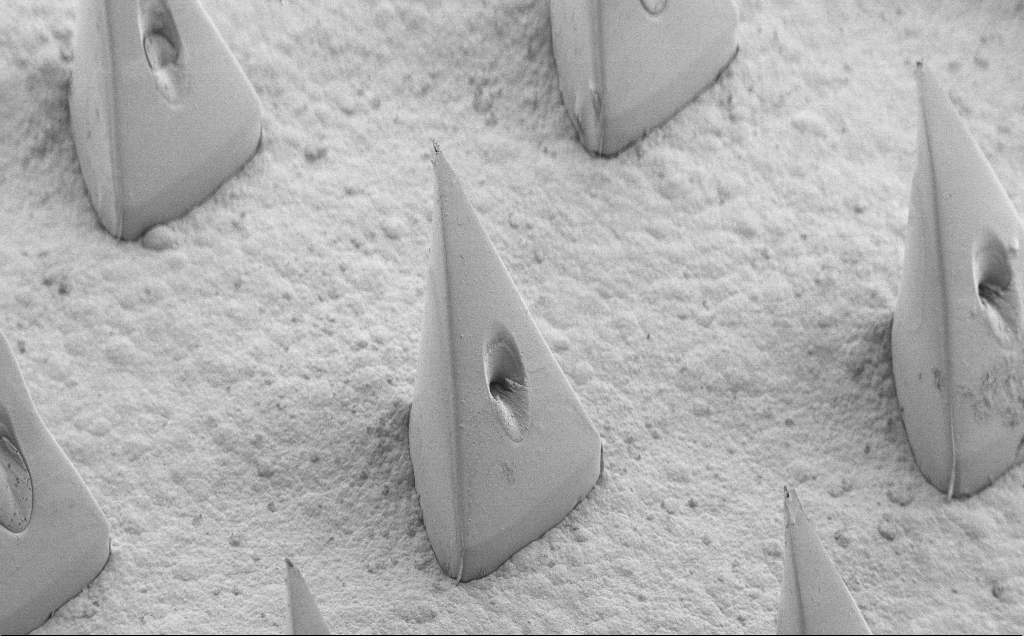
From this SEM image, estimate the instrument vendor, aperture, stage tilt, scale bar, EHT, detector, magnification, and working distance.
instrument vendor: Zeiss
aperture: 30 µm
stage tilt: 40°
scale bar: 200000 nm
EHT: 5 kV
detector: SE2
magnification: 0.123 K X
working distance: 9 mm